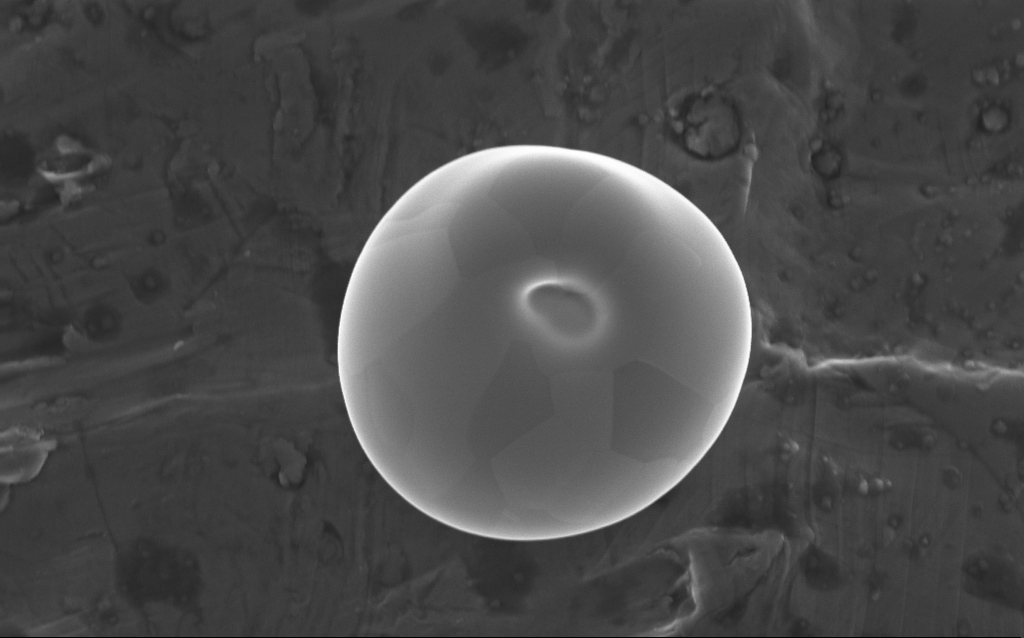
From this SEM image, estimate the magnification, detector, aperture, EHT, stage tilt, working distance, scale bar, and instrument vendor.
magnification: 54.73 K X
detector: InLens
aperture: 30 µm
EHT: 5 kV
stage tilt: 0°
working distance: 4 mm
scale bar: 1000 nm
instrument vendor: Zeiss